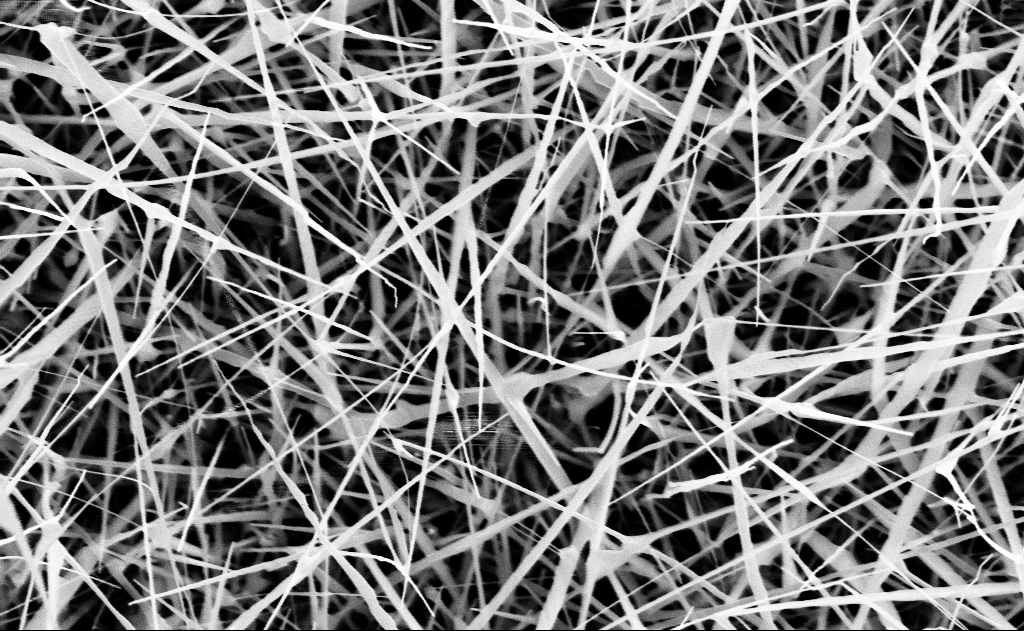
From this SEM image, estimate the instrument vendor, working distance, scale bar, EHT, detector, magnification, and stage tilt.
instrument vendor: Zeiss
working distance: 15 mm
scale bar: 1000 nm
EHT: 10 kV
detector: InLens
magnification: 40 K X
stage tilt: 0°